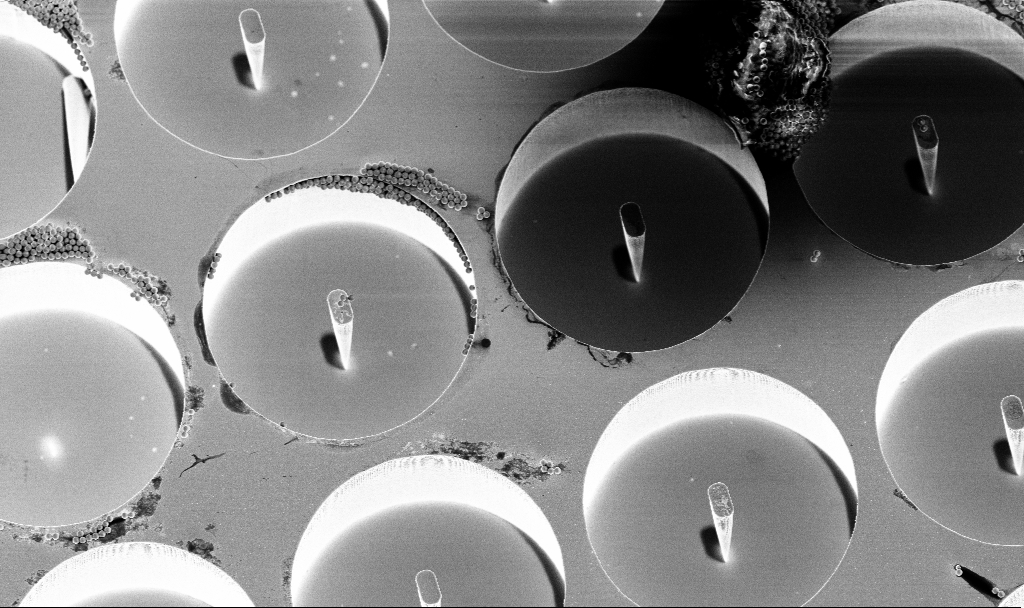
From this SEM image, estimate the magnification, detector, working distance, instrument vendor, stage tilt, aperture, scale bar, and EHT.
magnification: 3.41 K X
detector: InLens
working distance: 4.7 mm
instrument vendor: Zeiss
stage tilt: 15°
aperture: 30 µm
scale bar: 10000 nm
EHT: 4 kV